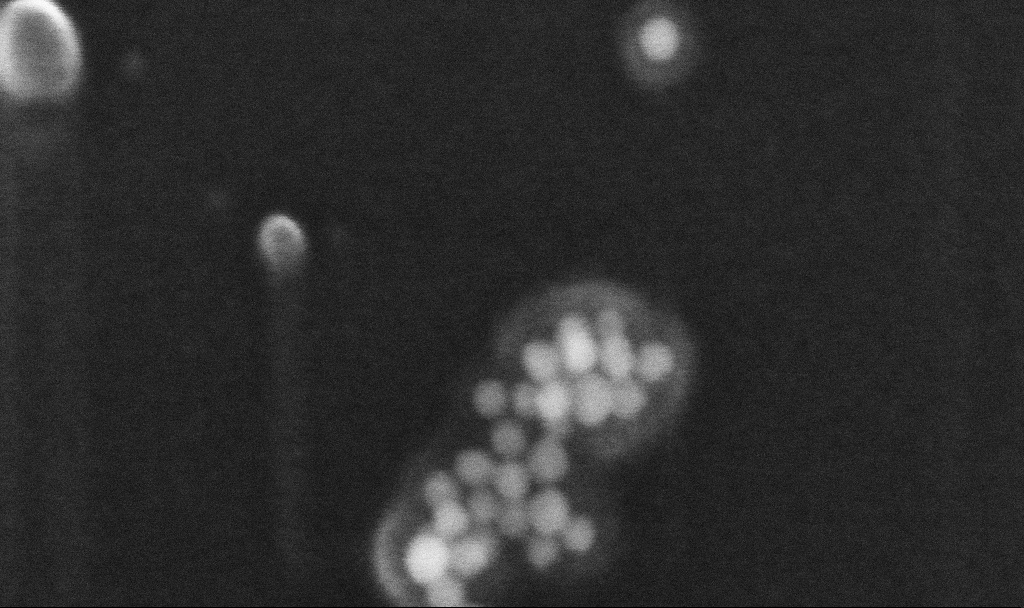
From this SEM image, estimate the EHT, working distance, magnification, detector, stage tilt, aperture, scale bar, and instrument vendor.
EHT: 10 kV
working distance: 3.2 mm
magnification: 704.13 K X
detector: InLens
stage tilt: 0°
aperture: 30 µm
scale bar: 100 nm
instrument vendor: Zeiss